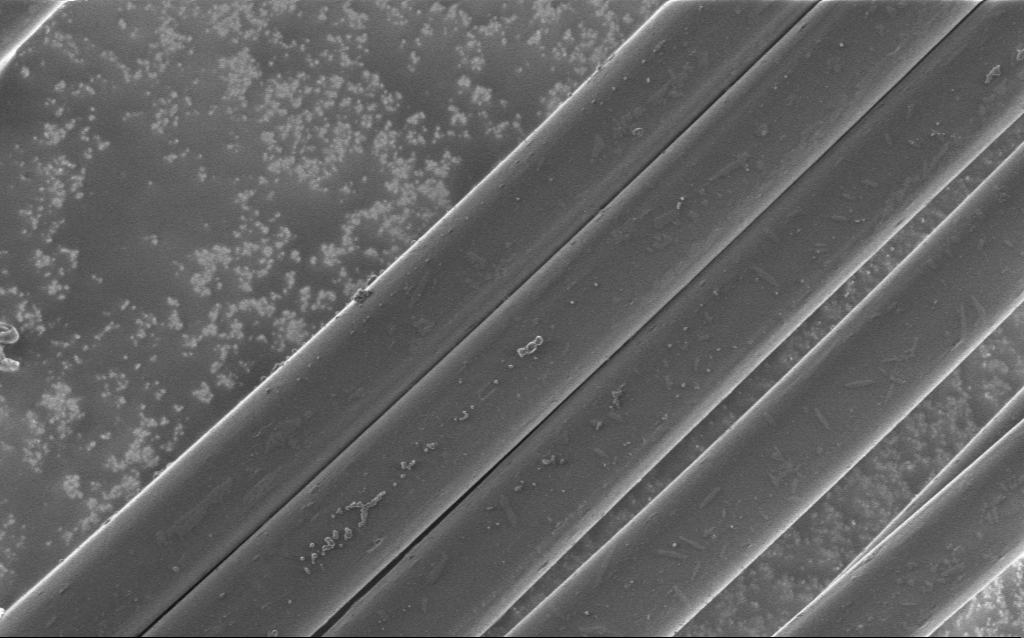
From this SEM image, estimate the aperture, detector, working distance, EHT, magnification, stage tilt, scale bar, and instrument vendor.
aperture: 30 µm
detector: InLens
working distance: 5 mm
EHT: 1 kV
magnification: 2.97 K X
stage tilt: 0°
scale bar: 20000 nm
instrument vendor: Zeiss